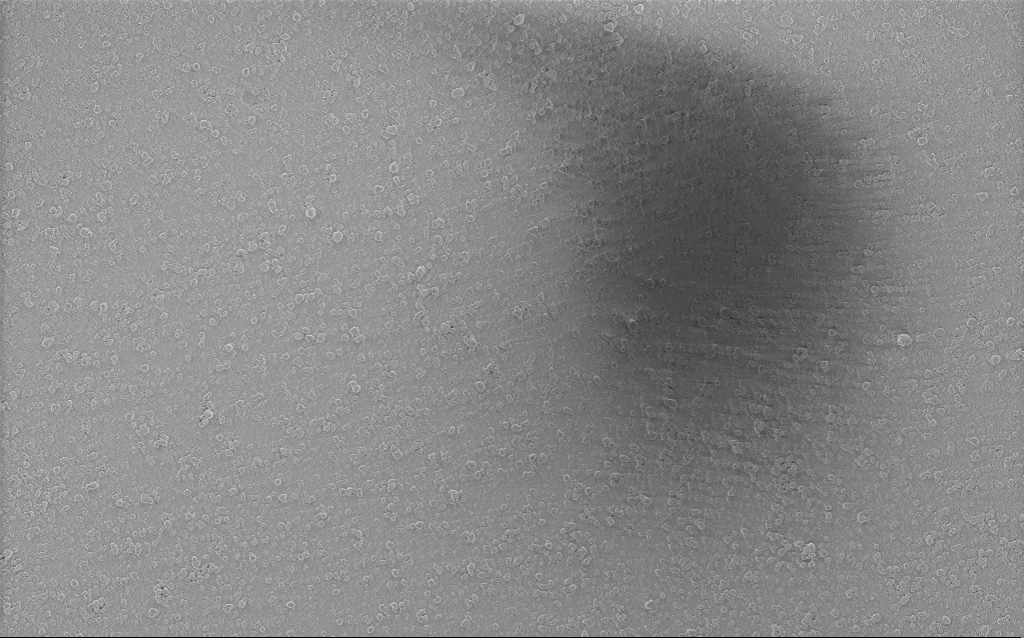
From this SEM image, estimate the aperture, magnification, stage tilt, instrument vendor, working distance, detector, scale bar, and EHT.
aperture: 20 µm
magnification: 0.235 K X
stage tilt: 0°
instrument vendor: Zeiss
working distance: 2.8 mm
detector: InLens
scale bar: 100000 nm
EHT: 3 kV